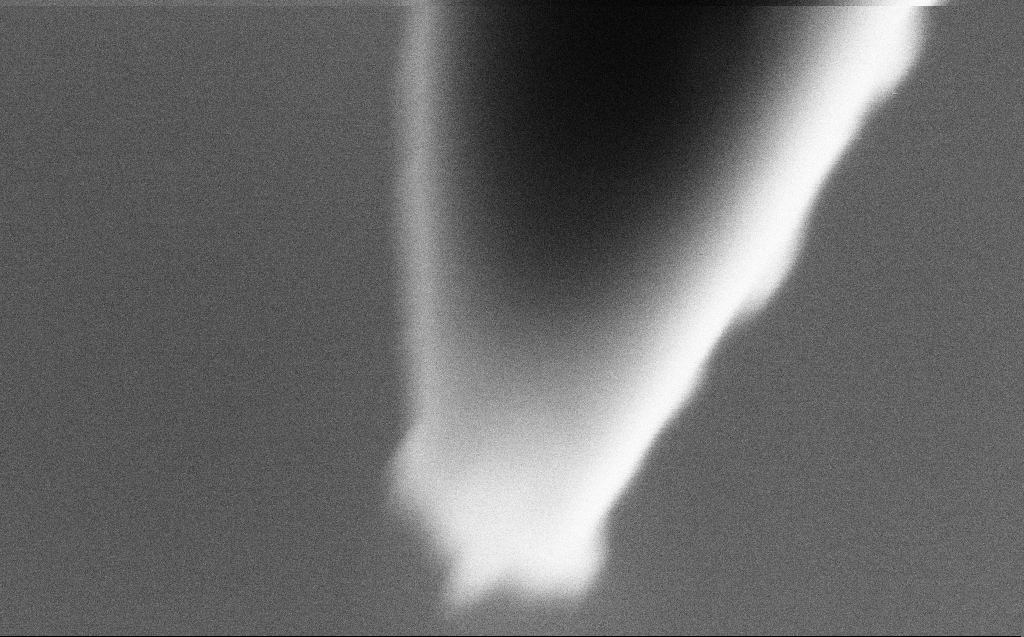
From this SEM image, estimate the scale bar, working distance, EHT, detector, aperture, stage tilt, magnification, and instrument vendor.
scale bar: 100 nm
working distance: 3 mm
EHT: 3 kV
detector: SE2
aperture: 30 µm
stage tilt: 45°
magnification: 647.43 K X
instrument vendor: Zeiss